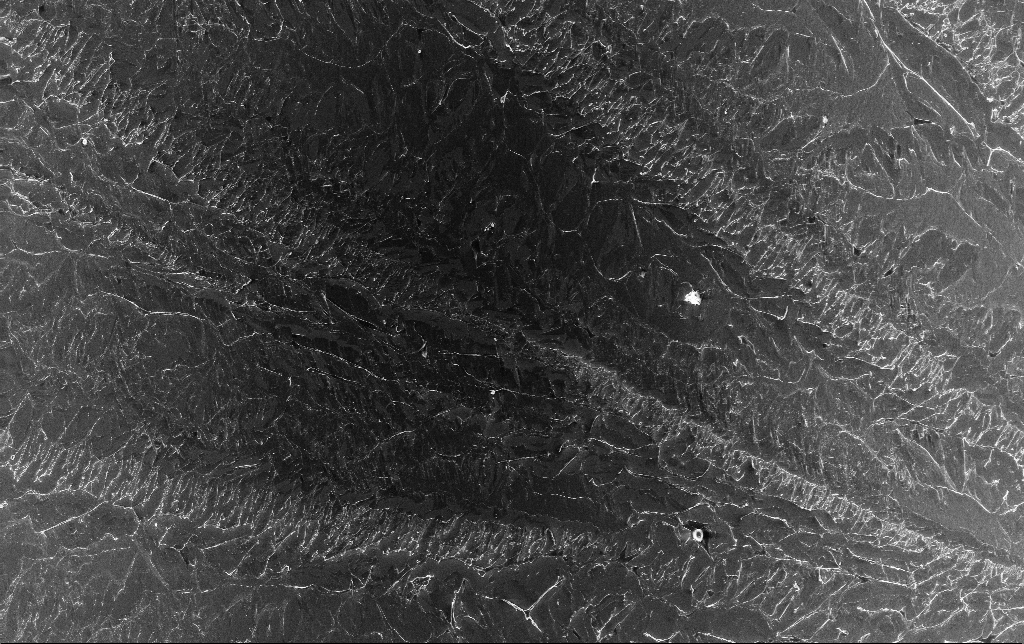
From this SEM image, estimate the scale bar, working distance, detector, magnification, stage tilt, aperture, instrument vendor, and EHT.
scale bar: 100000 nm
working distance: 3.2 mm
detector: InLens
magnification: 0.457 K X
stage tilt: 0°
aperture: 30 µm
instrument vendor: Zeiss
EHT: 10 kV